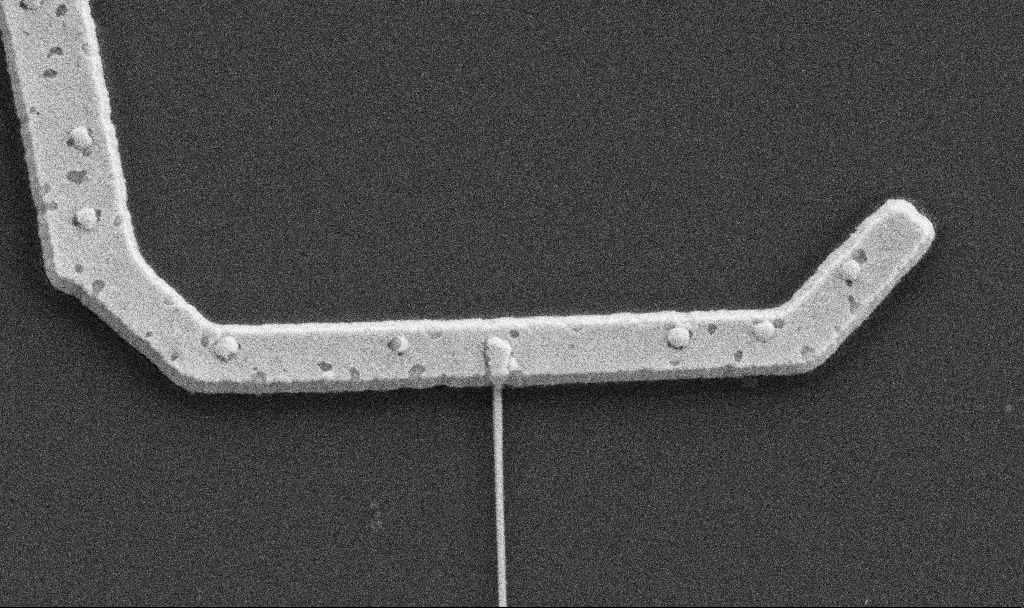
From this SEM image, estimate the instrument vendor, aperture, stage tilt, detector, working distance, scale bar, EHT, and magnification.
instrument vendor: Zeiss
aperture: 30 µm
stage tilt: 0°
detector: SE2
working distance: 10.6 mm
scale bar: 1000 nm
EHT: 5 kV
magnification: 43.77 K X